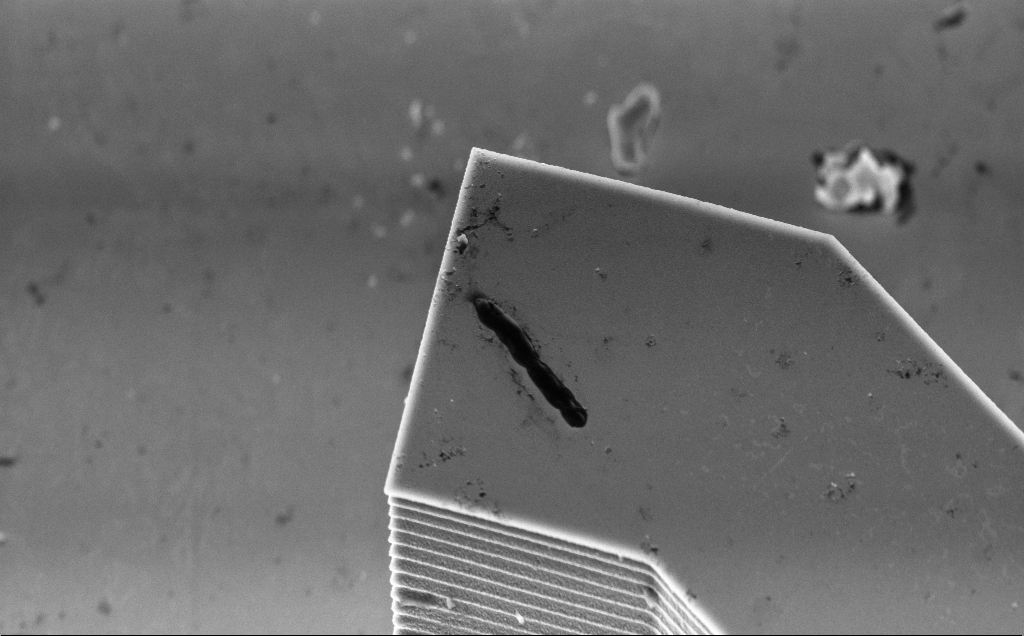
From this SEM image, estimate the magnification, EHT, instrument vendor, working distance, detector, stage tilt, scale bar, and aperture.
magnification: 12.98 K X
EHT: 5 kV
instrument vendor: Zeiss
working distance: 7 mm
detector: InLens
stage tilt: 45°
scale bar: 2000 nm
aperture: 30 µm